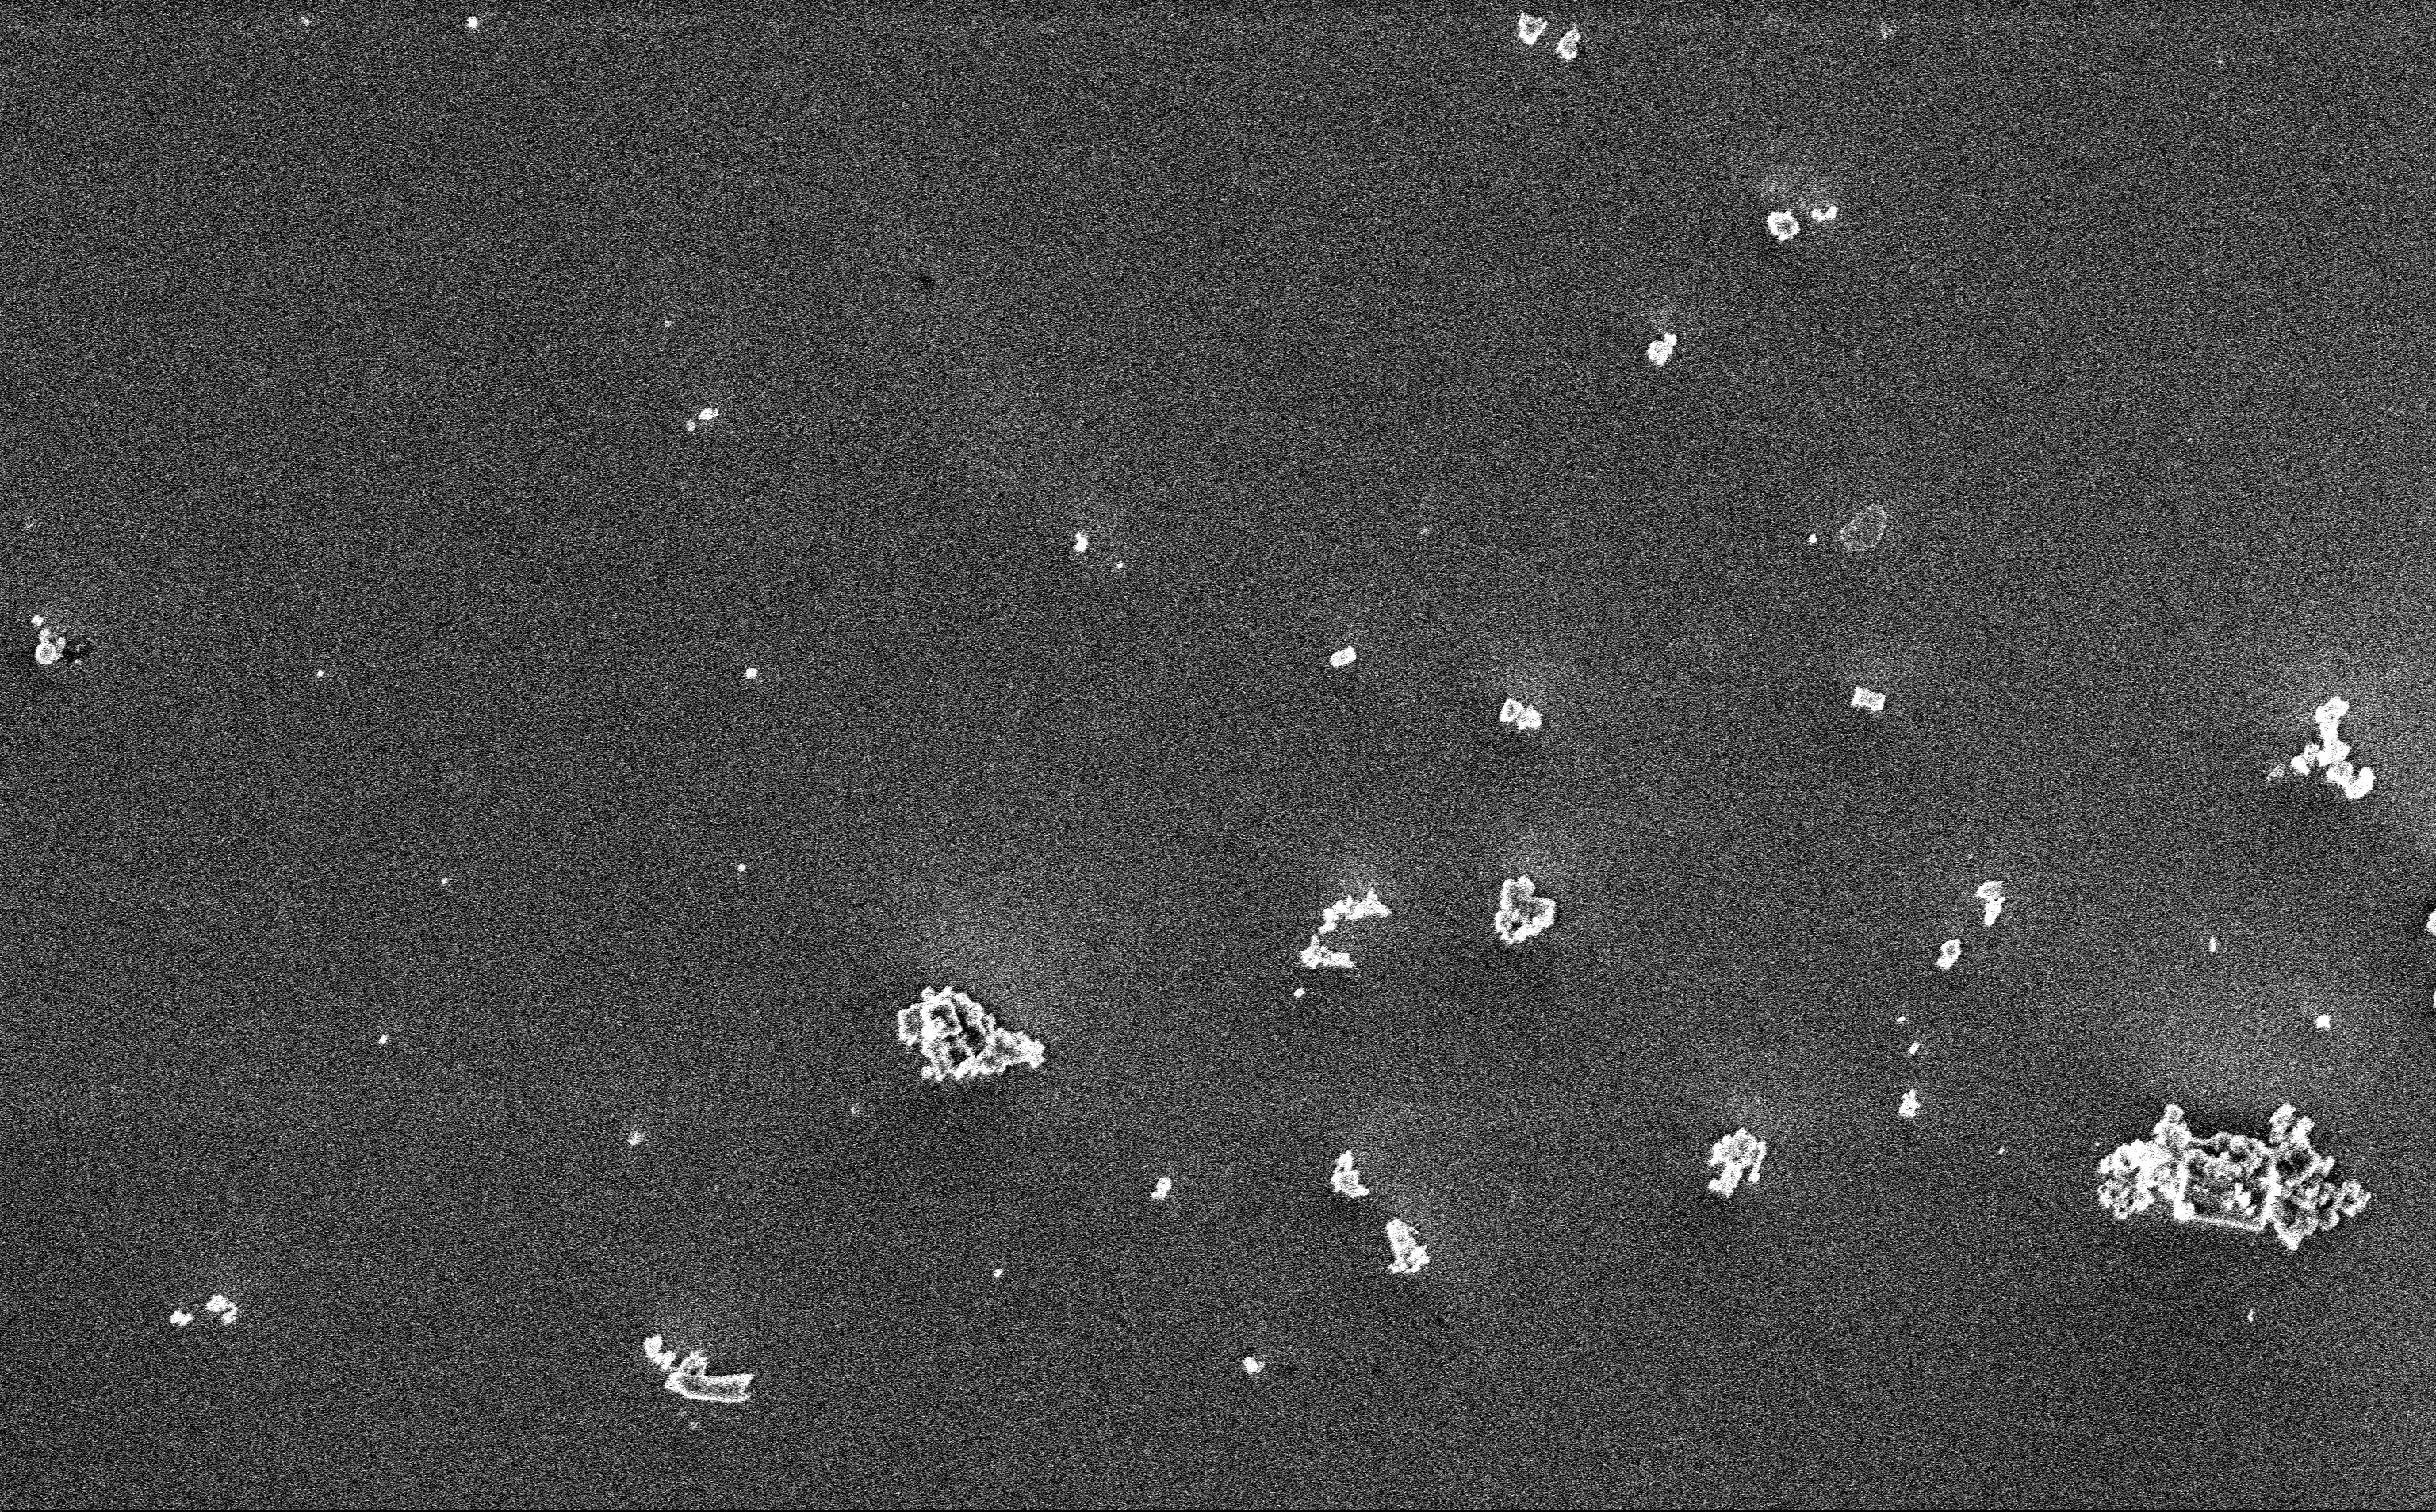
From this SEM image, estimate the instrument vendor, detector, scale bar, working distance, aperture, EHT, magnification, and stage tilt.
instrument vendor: Zeiss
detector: InLens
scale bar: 2000 nm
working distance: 3 mm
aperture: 30 µm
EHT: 3 kV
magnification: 12.85 K X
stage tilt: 0°